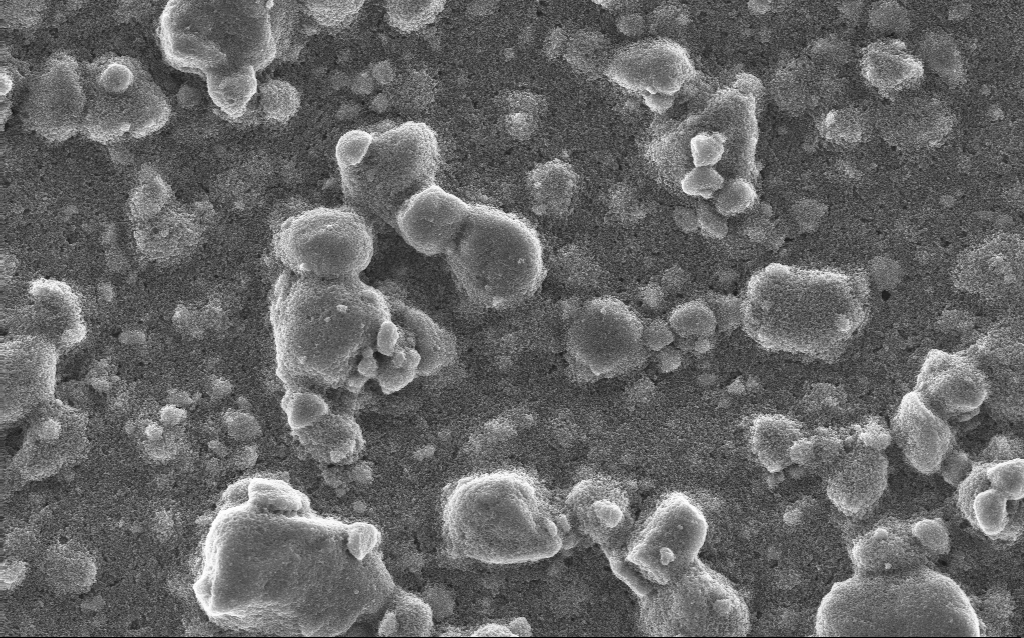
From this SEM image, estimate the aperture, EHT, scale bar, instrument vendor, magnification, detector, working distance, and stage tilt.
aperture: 30 µm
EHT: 10 kV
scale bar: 10000 nm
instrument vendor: Zeiss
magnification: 5 K X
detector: InLens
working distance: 2.7 mm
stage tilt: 0°